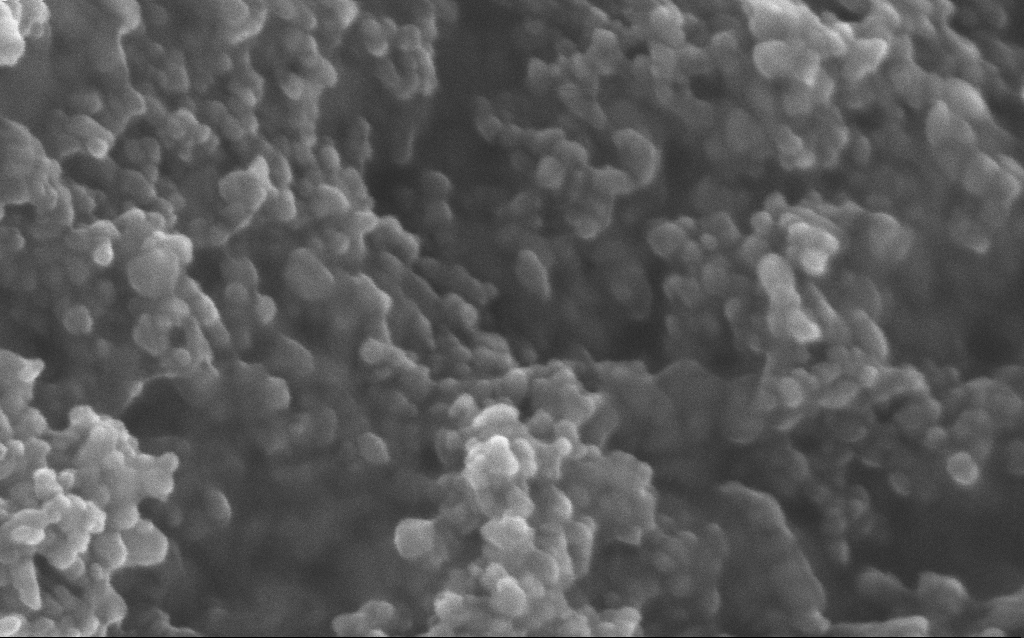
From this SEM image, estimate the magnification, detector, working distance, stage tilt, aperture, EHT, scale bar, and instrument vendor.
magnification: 348.1 K X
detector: InLens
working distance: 2.8 mm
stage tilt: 0°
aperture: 30 µm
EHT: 10 kV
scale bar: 100 nm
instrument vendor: Zeiss